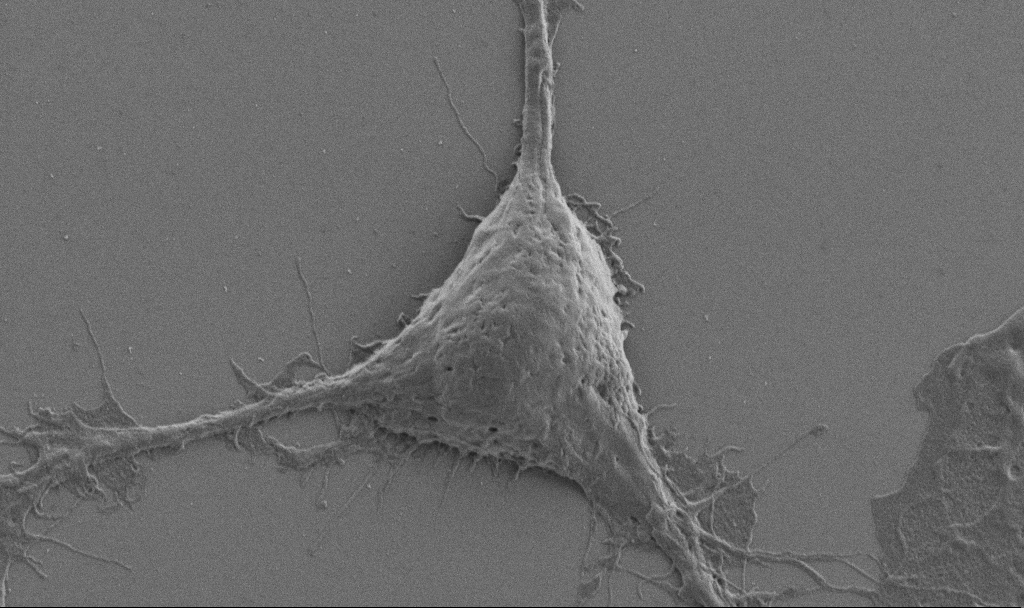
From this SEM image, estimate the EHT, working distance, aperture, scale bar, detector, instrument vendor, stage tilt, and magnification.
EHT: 1 kV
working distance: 6.9 mm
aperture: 30 µm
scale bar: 2000 nm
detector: SE2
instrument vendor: Zeiss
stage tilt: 0°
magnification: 10 K X